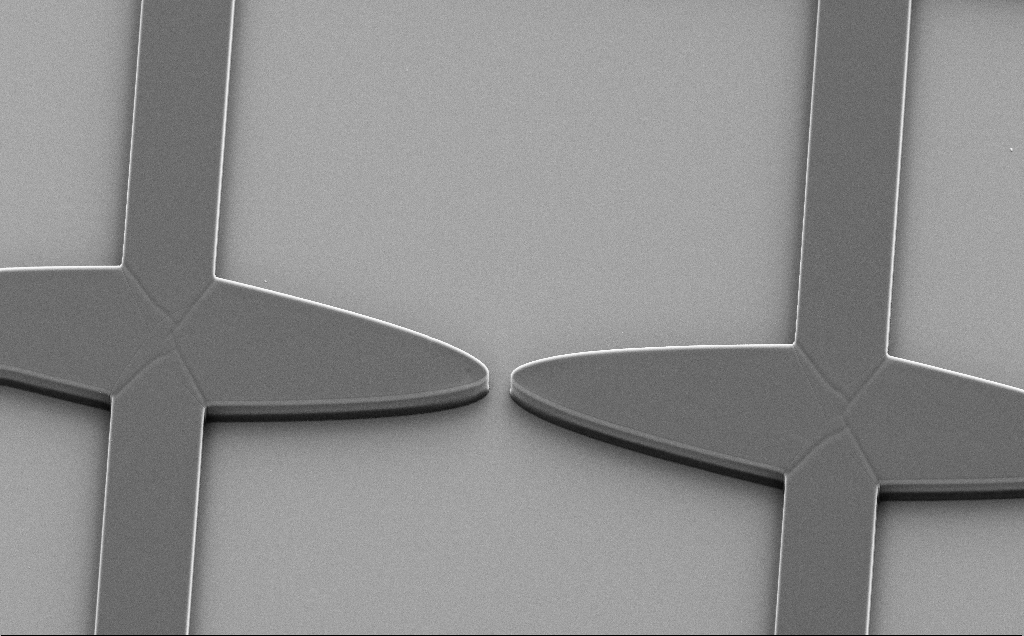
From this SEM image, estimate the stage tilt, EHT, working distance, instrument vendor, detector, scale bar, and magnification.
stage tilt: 35.3°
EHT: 10 kV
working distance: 11 mm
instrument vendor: Zeiss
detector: SE2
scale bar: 100000 nm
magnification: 0.69 K X